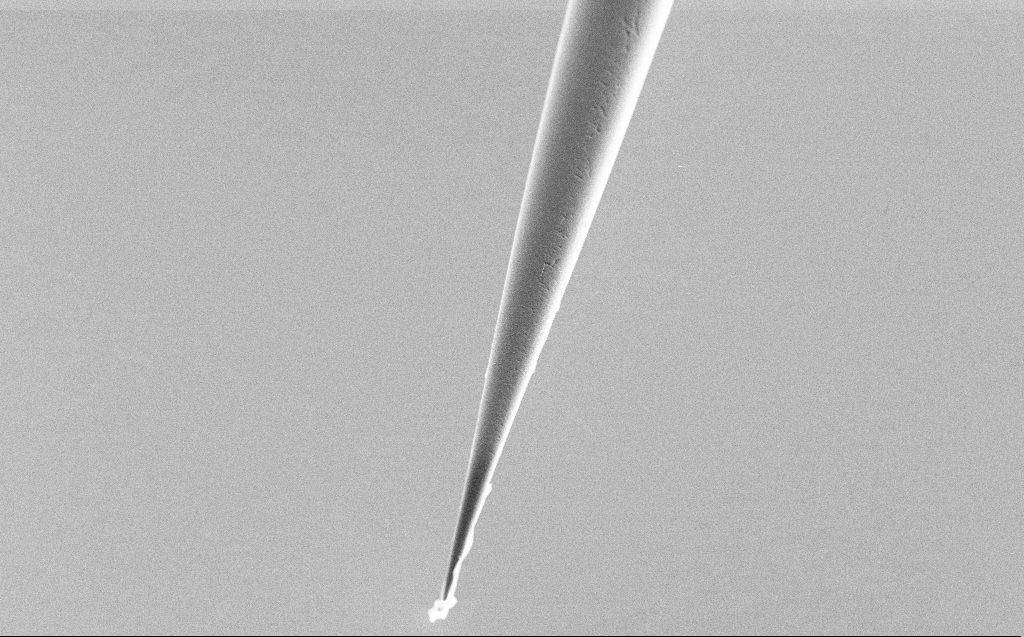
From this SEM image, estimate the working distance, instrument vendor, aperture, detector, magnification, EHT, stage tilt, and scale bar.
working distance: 6 mm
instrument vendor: Zeiss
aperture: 30 µm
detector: SE2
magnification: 2.5 K X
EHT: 5 kV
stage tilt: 45°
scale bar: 10000 nm